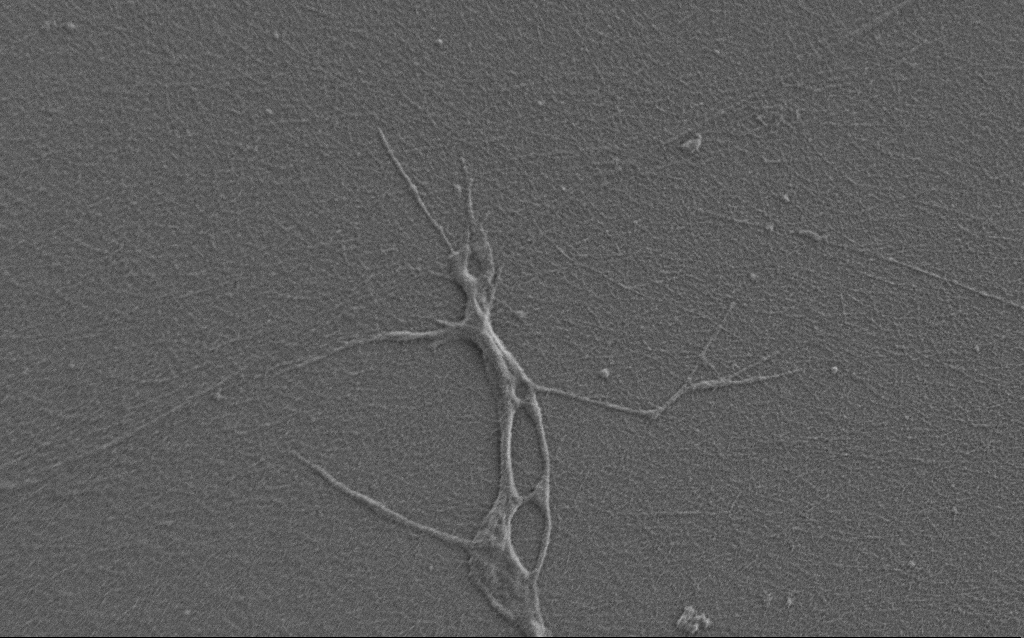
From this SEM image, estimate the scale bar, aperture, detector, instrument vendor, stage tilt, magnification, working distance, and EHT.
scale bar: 2000 nm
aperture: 30 µm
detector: SE2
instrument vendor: Zeiss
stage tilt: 0°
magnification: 7.5 K X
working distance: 7 mm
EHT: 0.9 kV